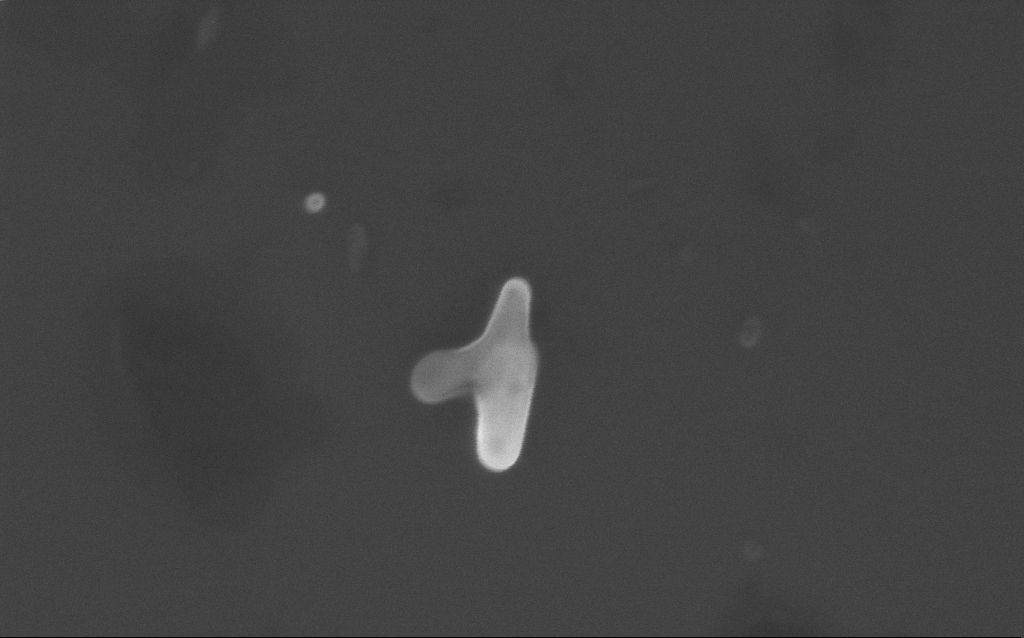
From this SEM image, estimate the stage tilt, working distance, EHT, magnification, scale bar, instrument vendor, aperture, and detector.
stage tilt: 0°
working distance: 3 mm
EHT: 10 kV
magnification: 131.01 K X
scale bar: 200 nm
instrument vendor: Zeiss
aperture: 30 µm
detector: InLens